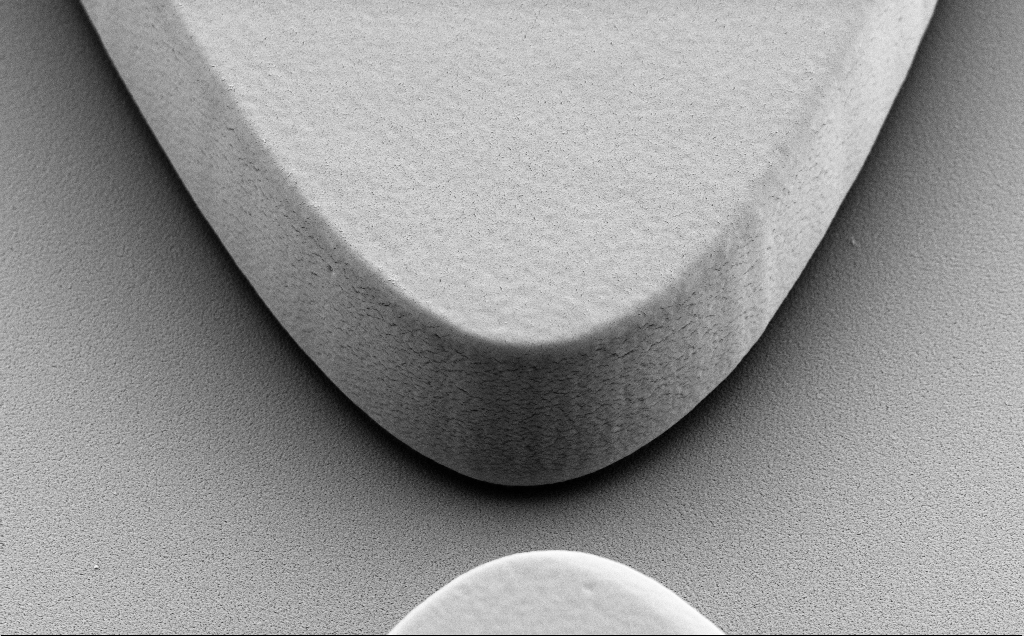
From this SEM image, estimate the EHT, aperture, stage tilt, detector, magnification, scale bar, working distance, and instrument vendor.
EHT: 5 kV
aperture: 30 µm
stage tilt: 40°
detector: SE2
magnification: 15 K X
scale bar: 1000 nm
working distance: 9 mm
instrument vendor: Zeiss